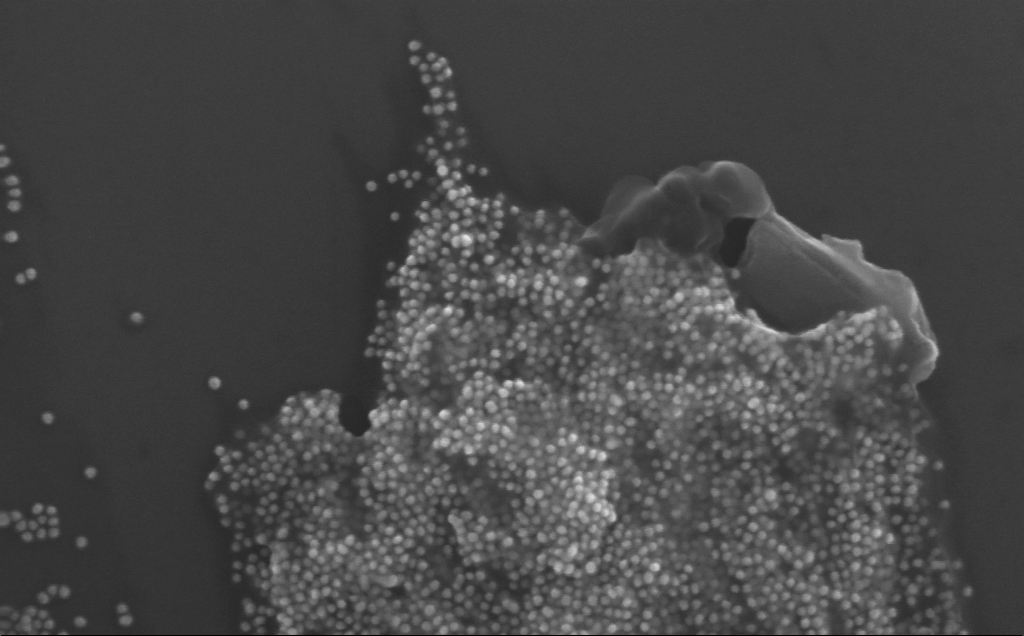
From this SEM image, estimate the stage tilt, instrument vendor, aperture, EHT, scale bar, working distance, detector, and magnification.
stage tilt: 0°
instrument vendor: Zeiss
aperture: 30 µm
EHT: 10 kV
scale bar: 100 nm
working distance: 3 mm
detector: InLens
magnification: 232.97 K X